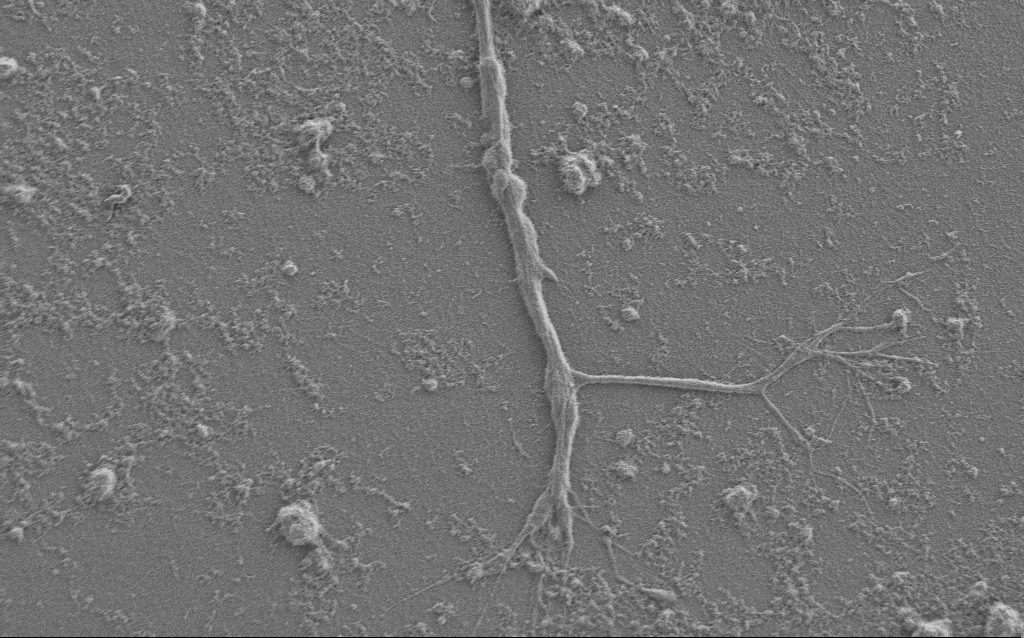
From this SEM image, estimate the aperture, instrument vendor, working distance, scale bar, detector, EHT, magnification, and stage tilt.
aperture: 30 µm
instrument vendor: Zeiss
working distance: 6 mm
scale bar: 10000 nm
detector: SE2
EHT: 1 kV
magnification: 5 K X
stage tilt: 0°